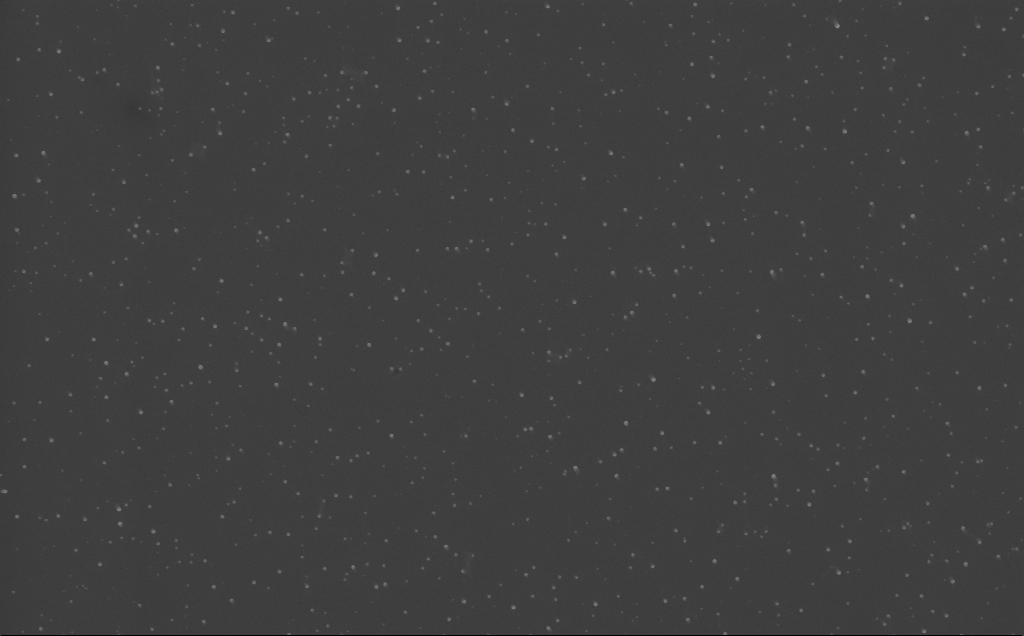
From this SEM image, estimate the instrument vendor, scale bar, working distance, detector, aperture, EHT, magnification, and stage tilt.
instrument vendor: Zeiss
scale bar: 2000 nm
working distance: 6 mm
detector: InLens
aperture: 30 µm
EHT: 10 kV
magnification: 20 K X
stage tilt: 0°